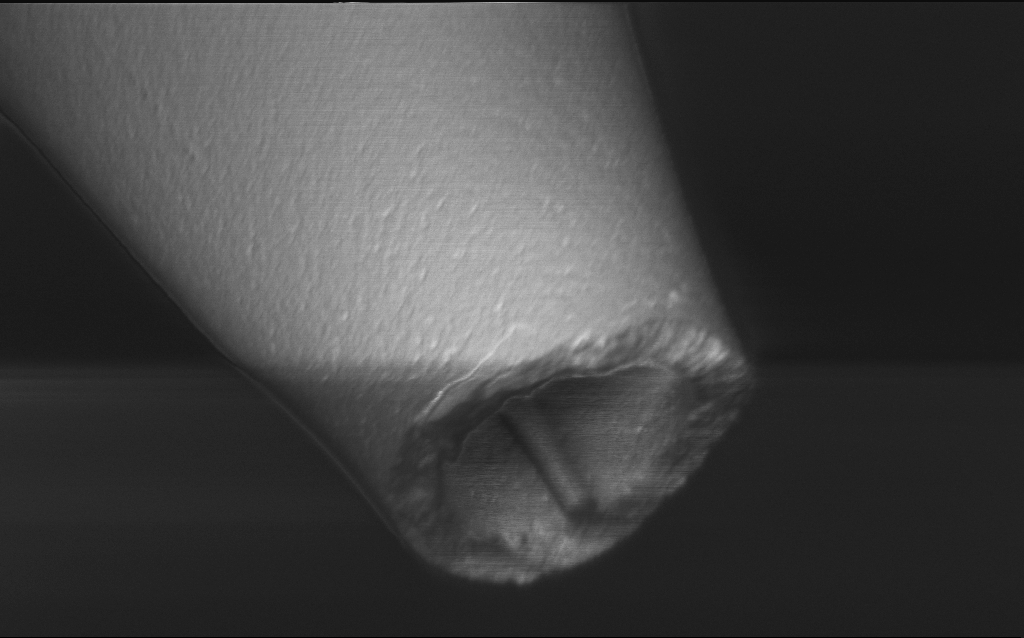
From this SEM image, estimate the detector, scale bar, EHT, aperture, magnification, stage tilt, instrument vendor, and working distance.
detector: InLens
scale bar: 1000 nm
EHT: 1 kV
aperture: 30 µm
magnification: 50 K X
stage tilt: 45°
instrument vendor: Zeiss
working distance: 7 mm